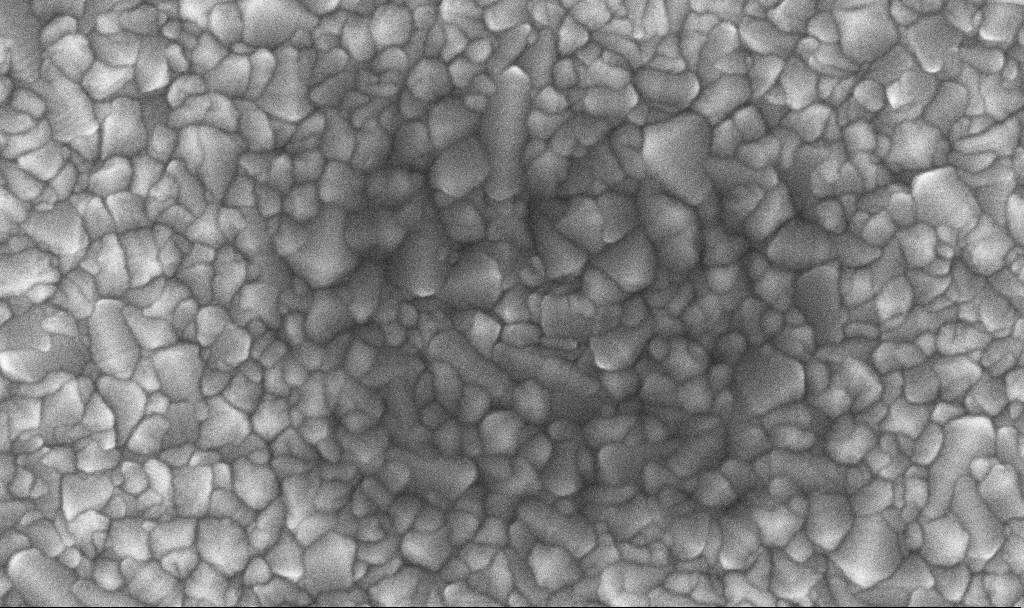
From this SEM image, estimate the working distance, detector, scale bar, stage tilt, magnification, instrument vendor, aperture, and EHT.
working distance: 1.6 mm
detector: InLens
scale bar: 100 nm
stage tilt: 0°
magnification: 142.13 K X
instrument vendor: Zeiss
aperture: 30 µm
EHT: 10 kV